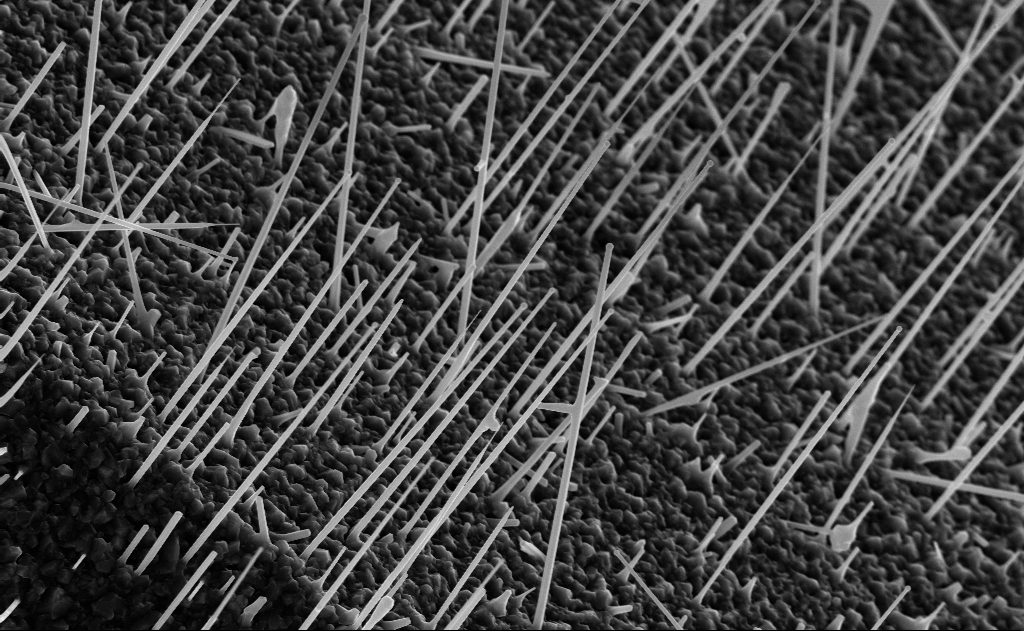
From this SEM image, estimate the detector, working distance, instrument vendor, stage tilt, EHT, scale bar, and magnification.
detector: InLens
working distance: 10 mm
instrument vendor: Zeiss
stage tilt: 0°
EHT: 10 kV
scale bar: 2000 nm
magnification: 20 K X